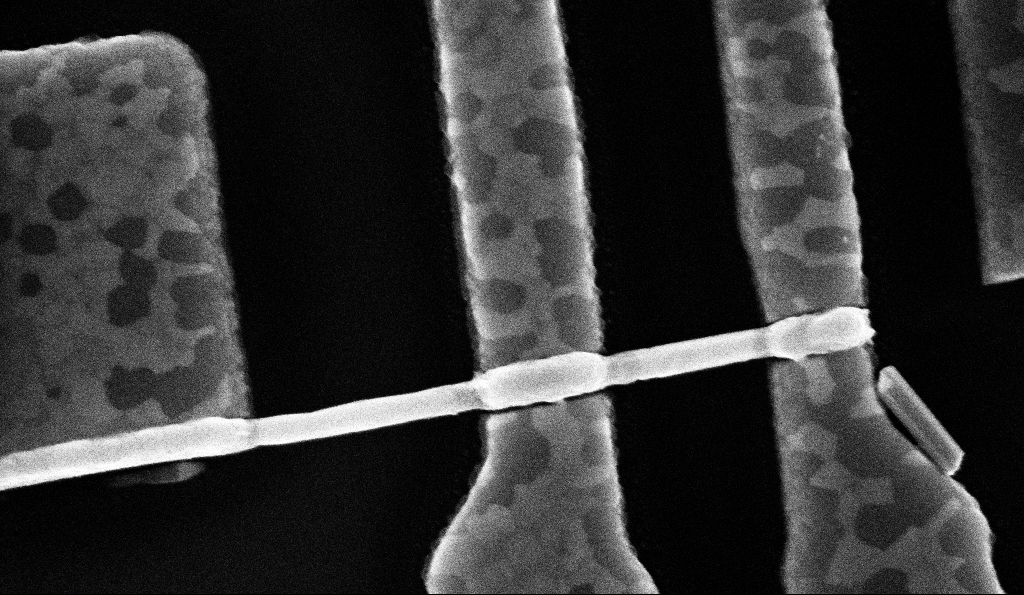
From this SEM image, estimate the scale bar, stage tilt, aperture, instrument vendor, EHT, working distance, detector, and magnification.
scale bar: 200 nm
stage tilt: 0°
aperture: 30 µm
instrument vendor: Zeiss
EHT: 10 kV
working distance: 8.5 mm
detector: InLens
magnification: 100 K X